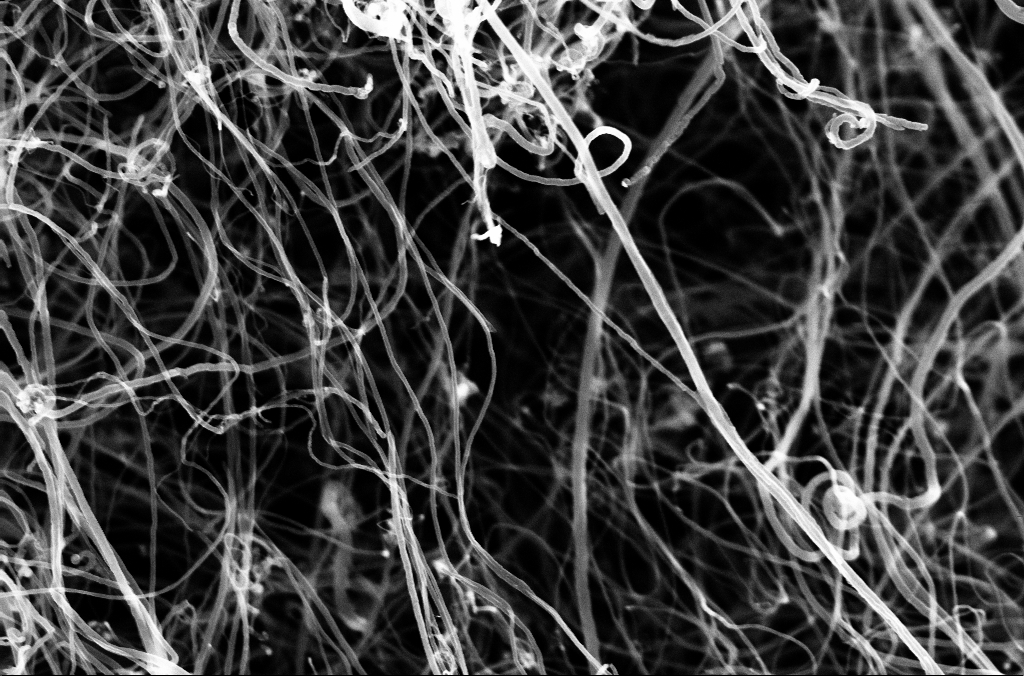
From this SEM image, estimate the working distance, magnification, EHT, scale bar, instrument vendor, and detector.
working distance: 3.3 mm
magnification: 51.87 K X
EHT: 10 kV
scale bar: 1000 nm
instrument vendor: Zeiss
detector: InLens